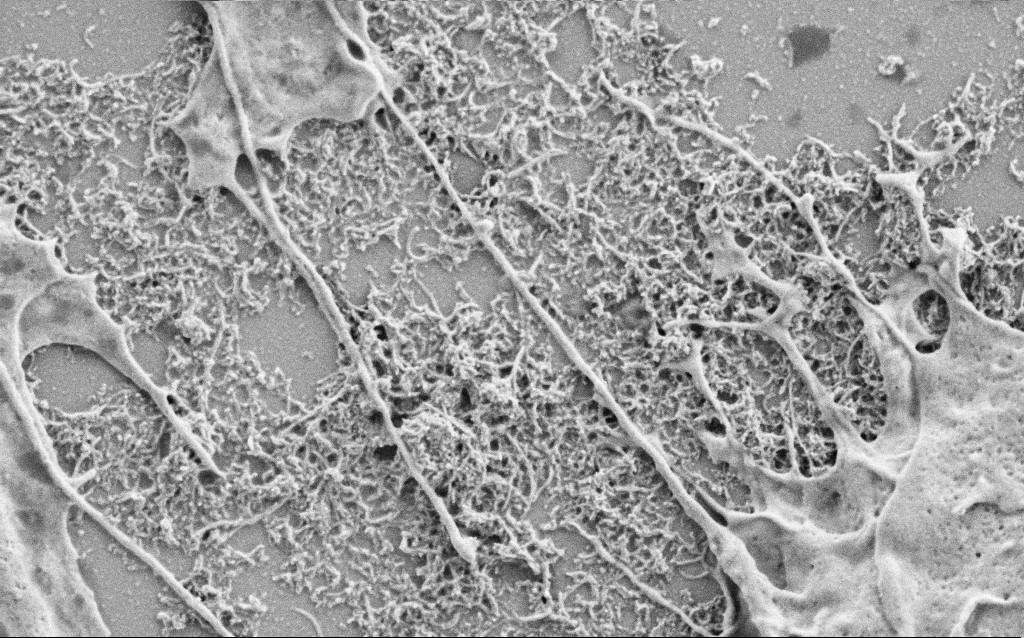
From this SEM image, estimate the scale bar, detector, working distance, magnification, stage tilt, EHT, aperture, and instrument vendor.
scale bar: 2000 nm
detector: SE2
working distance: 6.8 mm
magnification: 25 K X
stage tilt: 0°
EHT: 1.5 kV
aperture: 30 µm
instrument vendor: Zeiss